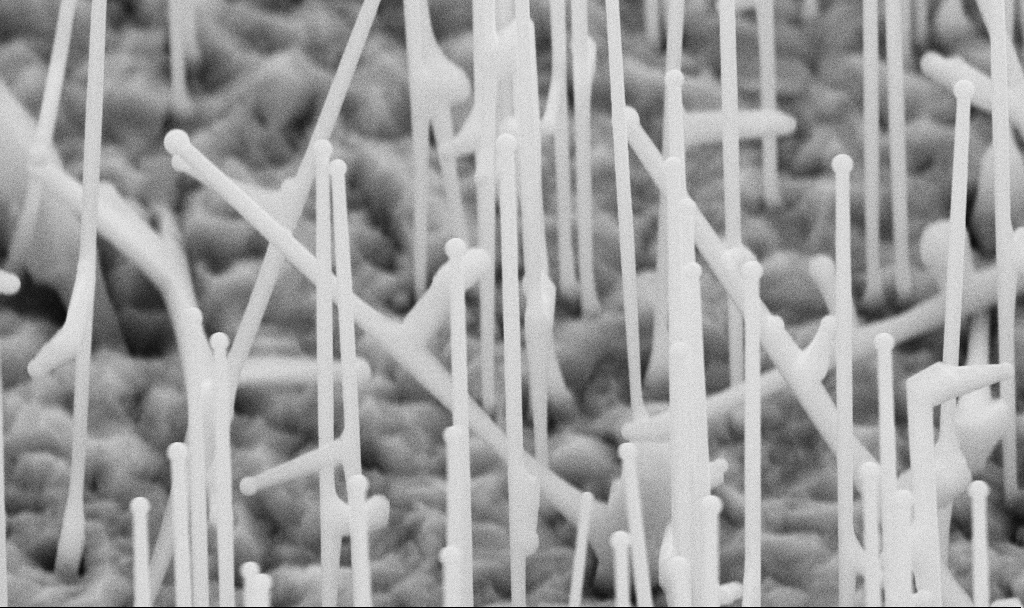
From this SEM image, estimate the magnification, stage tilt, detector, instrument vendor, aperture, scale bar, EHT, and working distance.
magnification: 50 K X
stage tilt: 45°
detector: SE2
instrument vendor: Zeiss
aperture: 30 µm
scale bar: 1000 nm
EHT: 10 kV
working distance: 6.7 mm